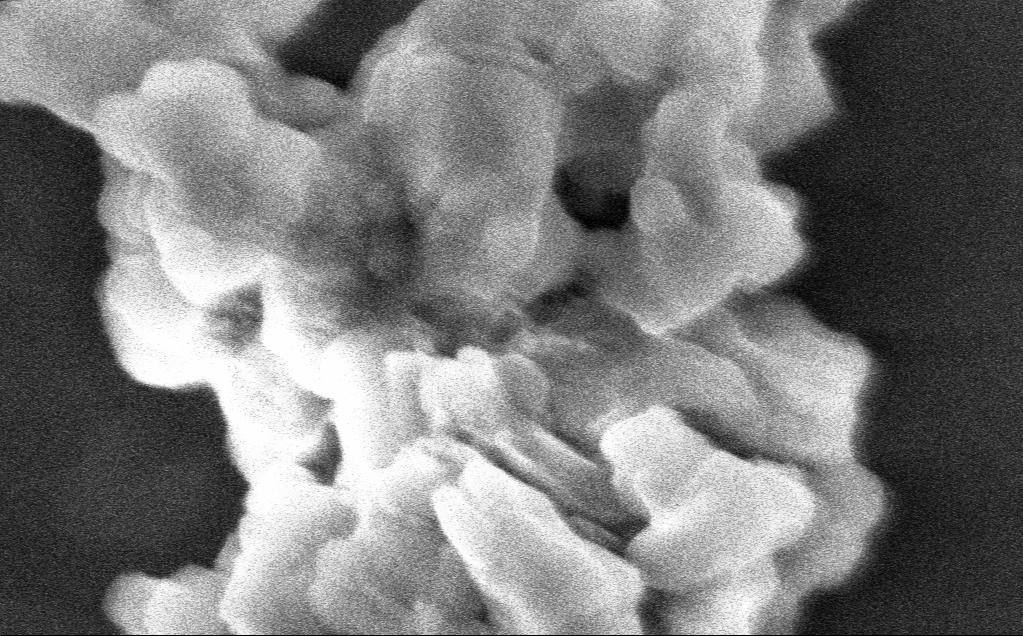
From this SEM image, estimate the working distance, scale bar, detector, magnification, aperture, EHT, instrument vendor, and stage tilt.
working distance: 3 mm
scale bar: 200 nm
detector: InLens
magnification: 286.95 K X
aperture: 30 µm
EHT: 3 kV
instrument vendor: Zeiss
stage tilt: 0°